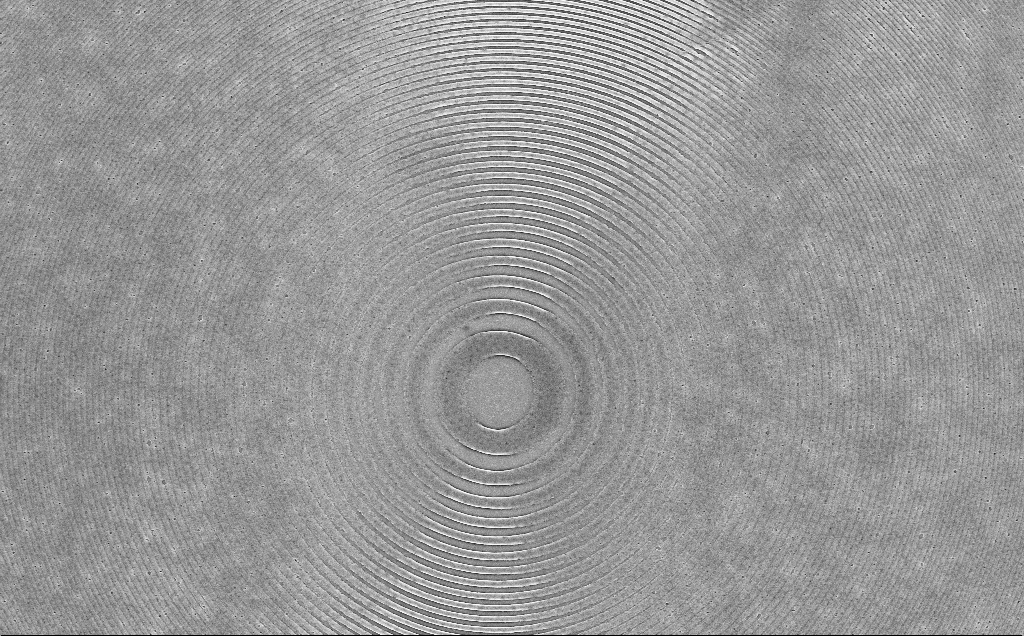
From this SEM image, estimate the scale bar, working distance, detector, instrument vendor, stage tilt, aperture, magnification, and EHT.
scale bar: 10000 nm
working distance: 7 mm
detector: InLens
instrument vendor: Zeiss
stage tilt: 0°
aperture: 30 µm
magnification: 3.75 K X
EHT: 5 kV